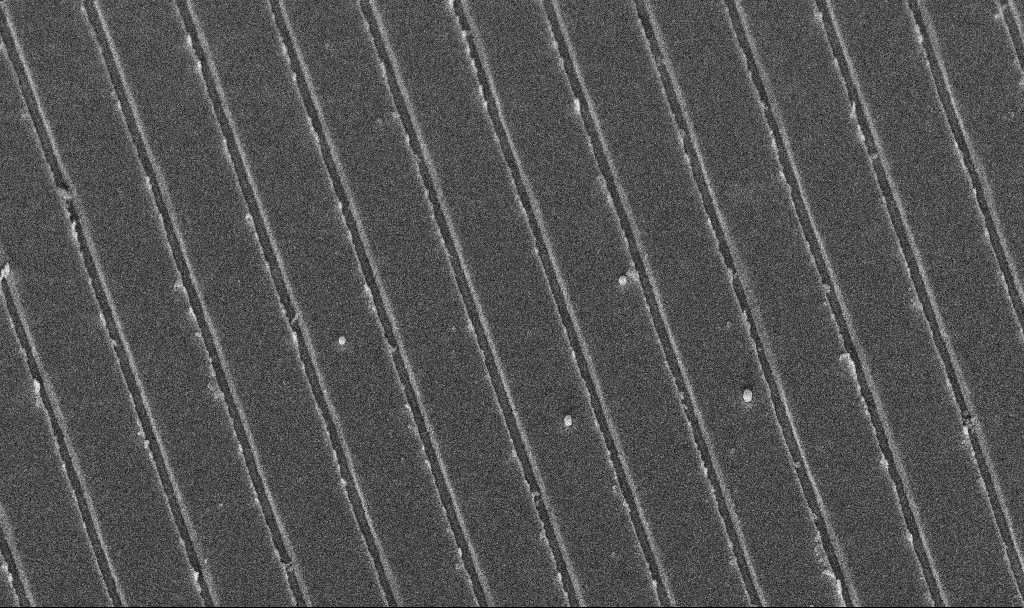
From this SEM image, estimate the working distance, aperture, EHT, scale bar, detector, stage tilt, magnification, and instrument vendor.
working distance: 10.8 mm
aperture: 30 µm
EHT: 5 kV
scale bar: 1000 nm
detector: InLens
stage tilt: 45°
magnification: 38.99 K X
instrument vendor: Zeiss